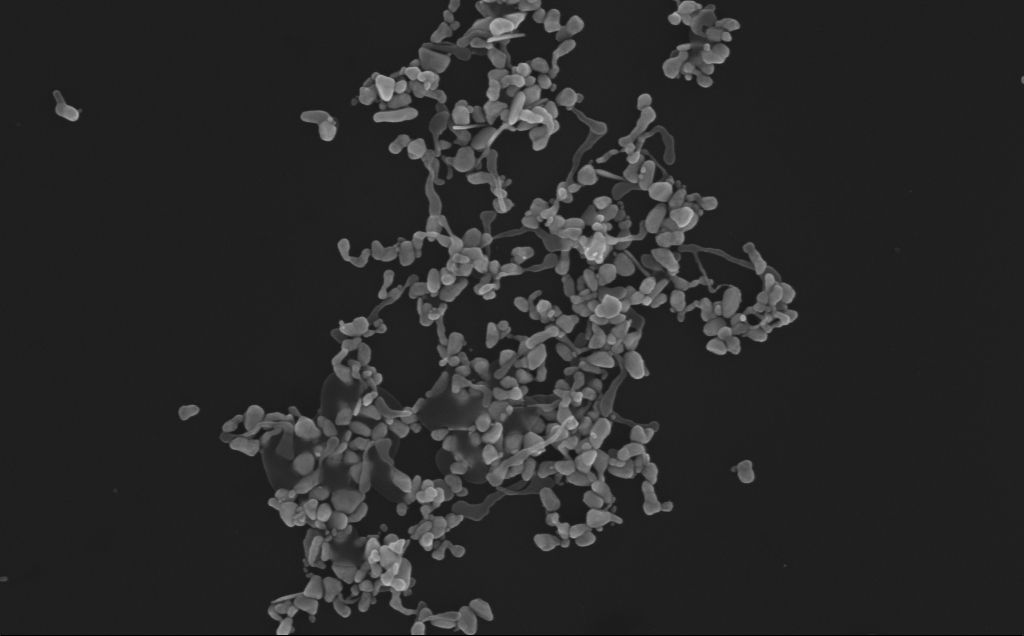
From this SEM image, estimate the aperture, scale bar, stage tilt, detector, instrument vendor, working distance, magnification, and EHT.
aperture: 30 µm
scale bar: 200 nm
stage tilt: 0°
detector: InLens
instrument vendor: Zeiss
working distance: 3 mm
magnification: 101.81 K X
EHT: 10 kV